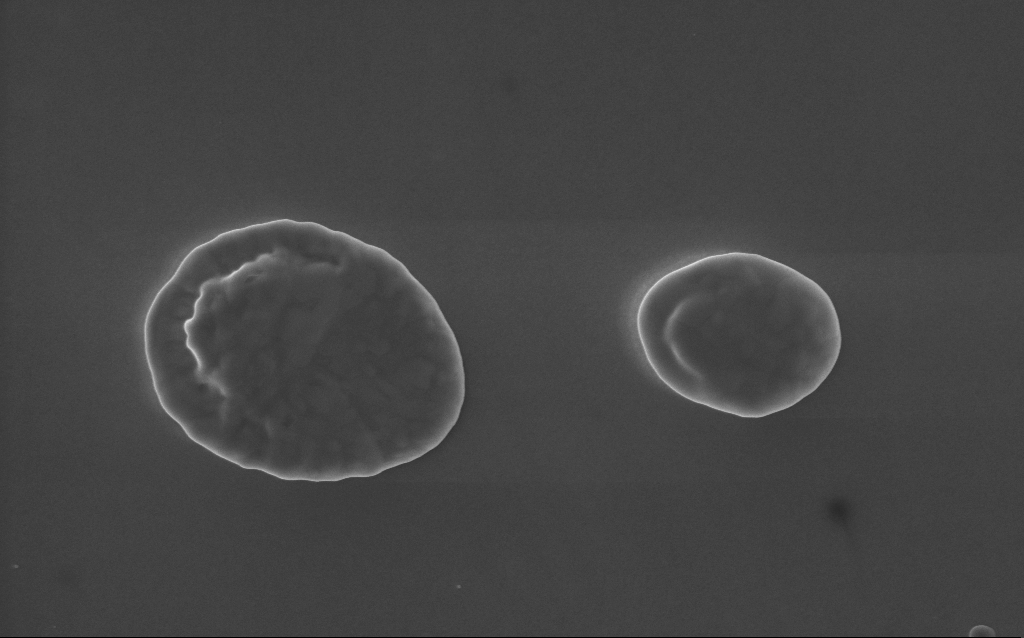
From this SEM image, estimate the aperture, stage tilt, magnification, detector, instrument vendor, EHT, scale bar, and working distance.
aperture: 30 µm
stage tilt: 0°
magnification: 58 K X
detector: InLens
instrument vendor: Zeiss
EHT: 5 kV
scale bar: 1000 nm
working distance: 3 mm